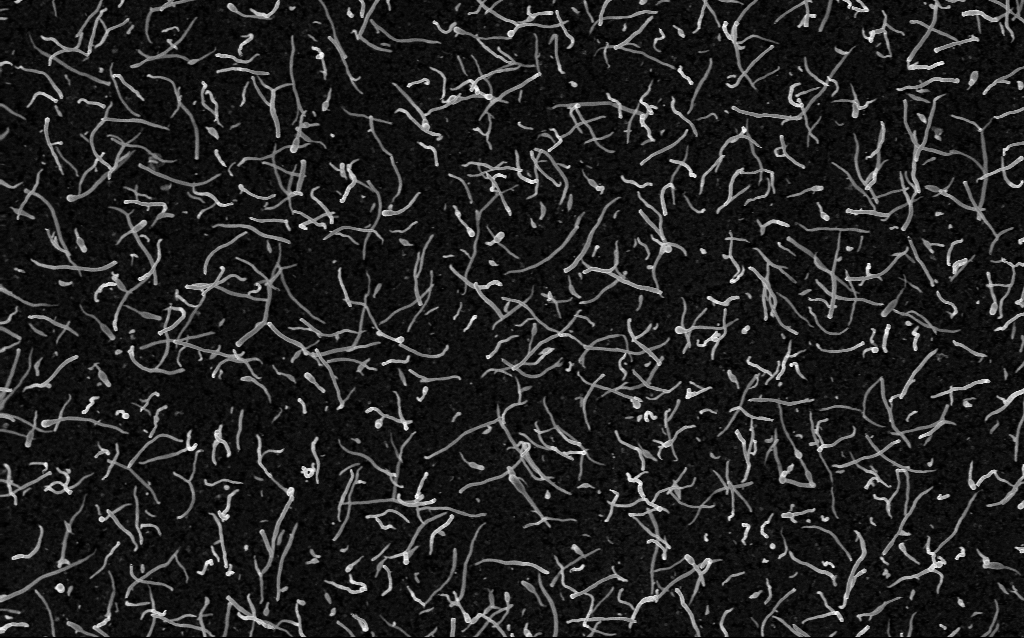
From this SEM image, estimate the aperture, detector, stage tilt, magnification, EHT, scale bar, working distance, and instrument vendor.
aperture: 30 µm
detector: InLens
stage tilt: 0°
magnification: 20 K X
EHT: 5 kV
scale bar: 1000 nm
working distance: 1.8 mm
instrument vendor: Zeiss